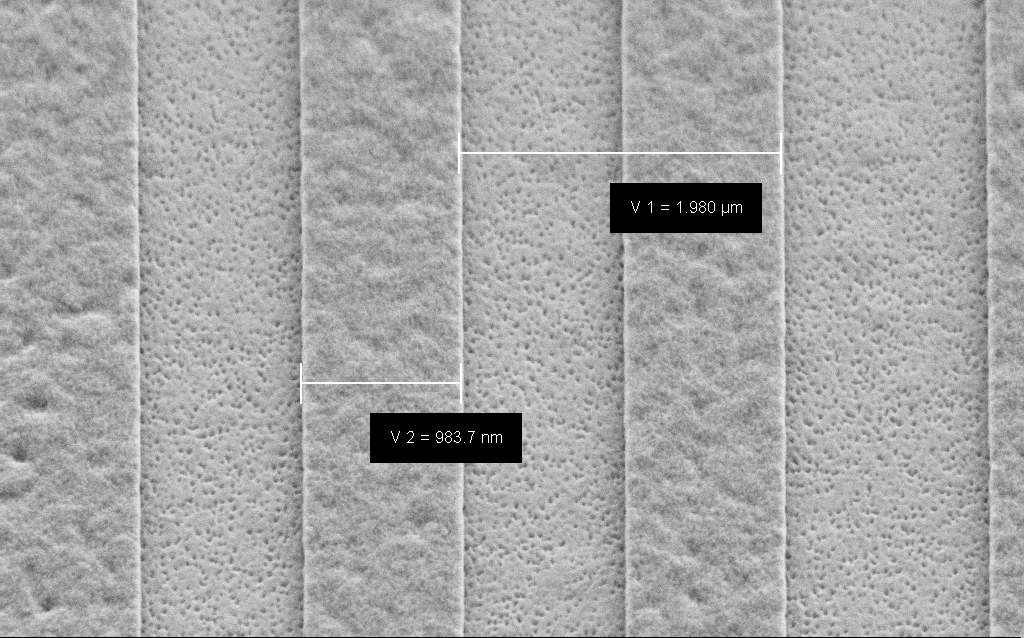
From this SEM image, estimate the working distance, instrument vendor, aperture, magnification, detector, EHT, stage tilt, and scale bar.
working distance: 6.6 mm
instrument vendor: Zeiss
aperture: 30 µm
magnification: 59.73 K X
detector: SE2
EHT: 3 kV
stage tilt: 45°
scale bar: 1000 nm